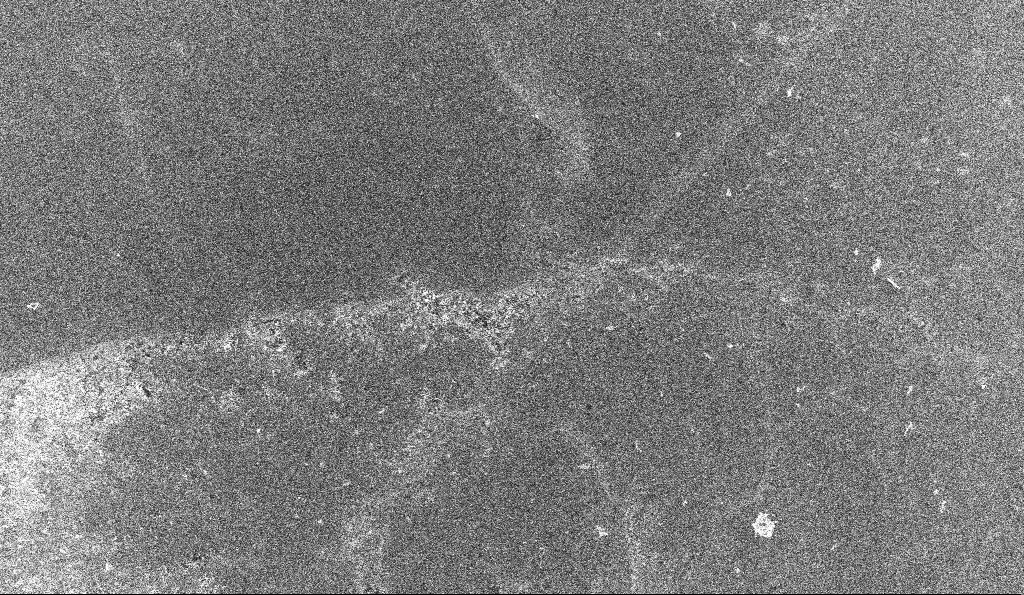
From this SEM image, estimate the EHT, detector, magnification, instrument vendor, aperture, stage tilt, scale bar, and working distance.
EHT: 10 kV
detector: InLens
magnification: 0.663 K X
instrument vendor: Zeiss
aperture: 30 µm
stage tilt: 0°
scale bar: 100000 nm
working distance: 5.2 mm